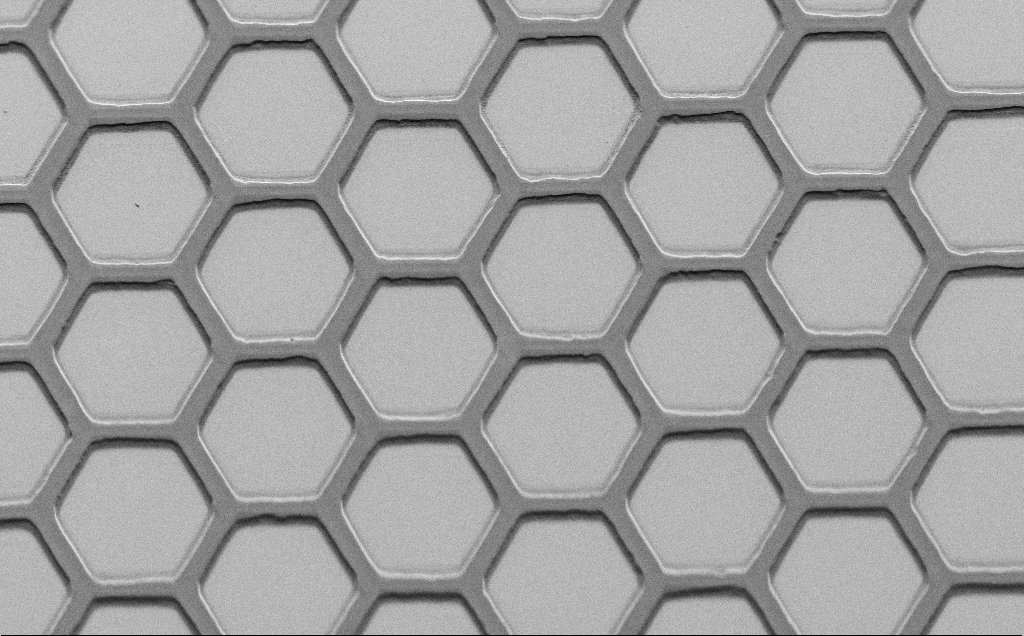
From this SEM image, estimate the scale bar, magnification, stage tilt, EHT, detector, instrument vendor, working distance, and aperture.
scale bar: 20000 nm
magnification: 1.01 K X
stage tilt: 45°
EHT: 1.5 kV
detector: SE2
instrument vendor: Zeiss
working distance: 7 mm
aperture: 30 µm